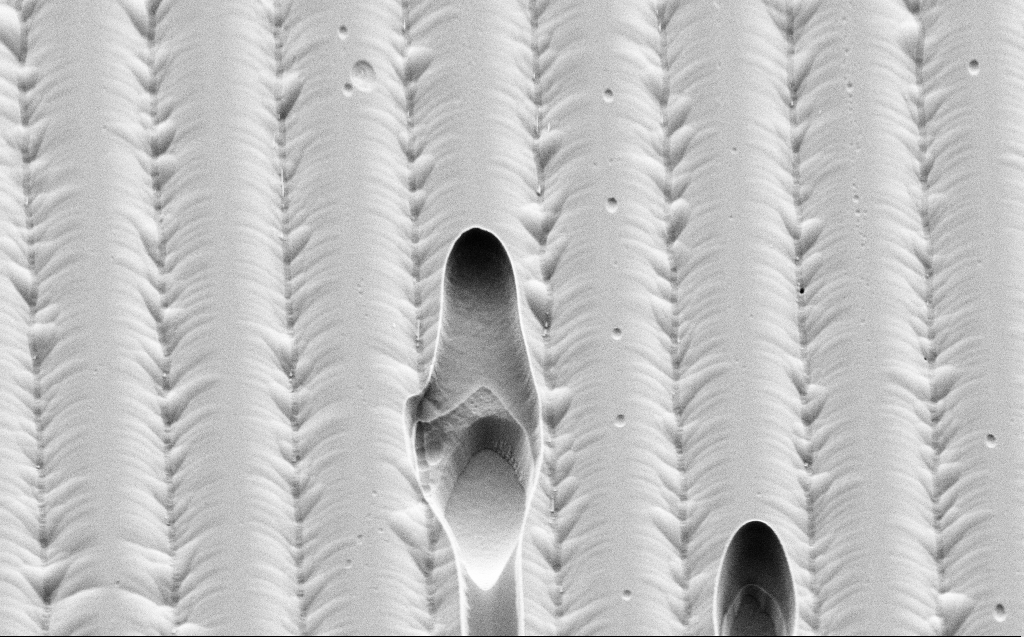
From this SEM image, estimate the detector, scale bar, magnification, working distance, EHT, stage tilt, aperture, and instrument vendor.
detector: SE2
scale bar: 10000 nm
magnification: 4.69 K X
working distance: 8 mm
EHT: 3 kV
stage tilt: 45°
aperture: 30 µm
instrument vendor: Zeiss